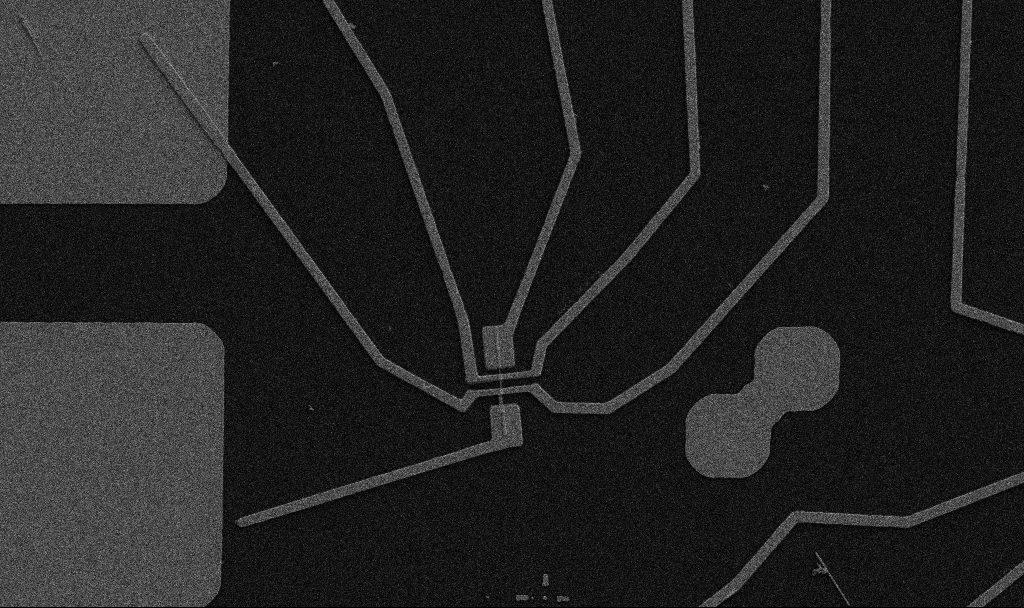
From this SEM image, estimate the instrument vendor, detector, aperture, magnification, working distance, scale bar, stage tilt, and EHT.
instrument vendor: Zeiss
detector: SE2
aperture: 30 µm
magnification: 5 K X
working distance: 10.7 mm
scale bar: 10000 nm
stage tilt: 0°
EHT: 5 kV